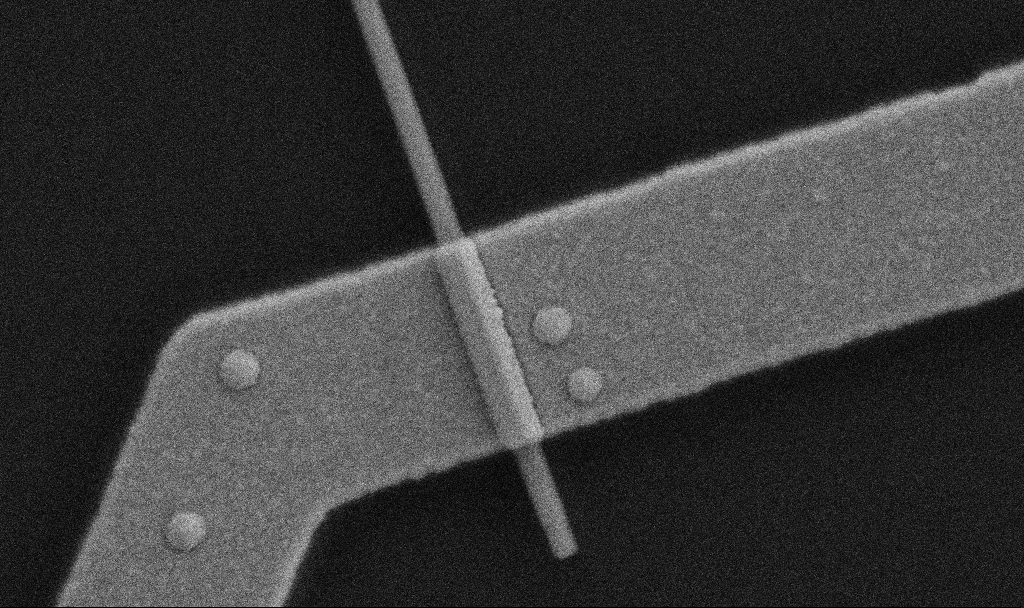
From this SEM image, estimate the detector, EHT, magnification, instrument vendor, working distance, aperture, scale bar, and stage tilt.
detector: SE2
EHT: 5 kV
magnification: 100 K X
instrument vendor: Zeiss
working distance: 10.7 mm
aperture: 30 µm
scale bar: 200 nm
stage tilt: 0°